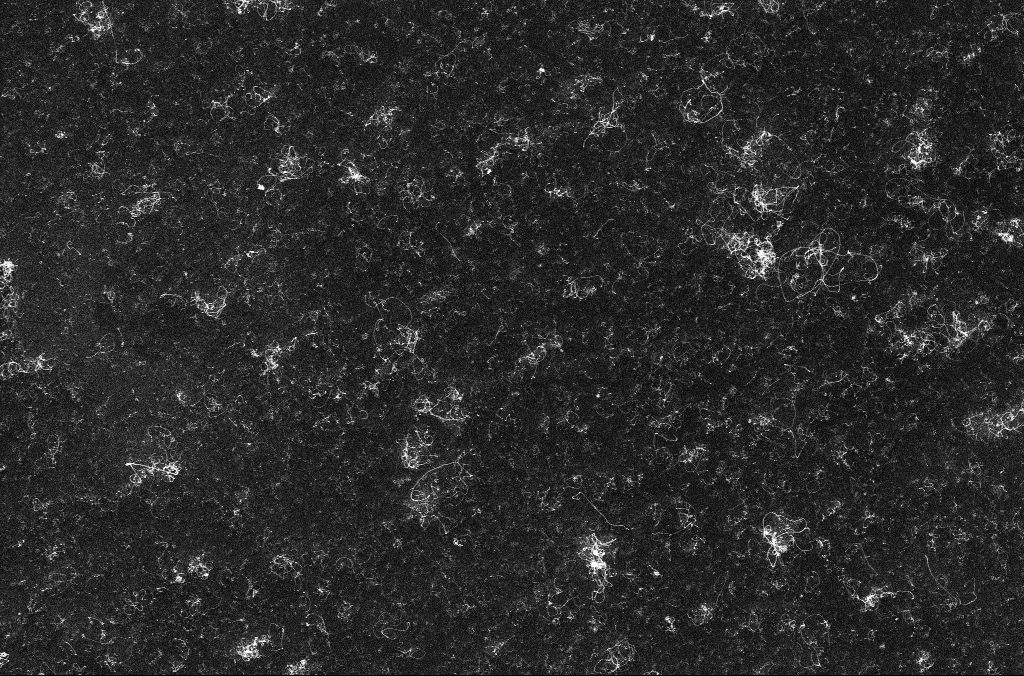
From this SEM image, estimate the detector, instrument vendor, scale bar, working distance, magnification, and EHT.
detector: InLens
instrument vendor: Zeiss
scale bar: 10000 nm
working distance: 3.3 mm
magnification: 4.26 K X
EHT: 10 kV